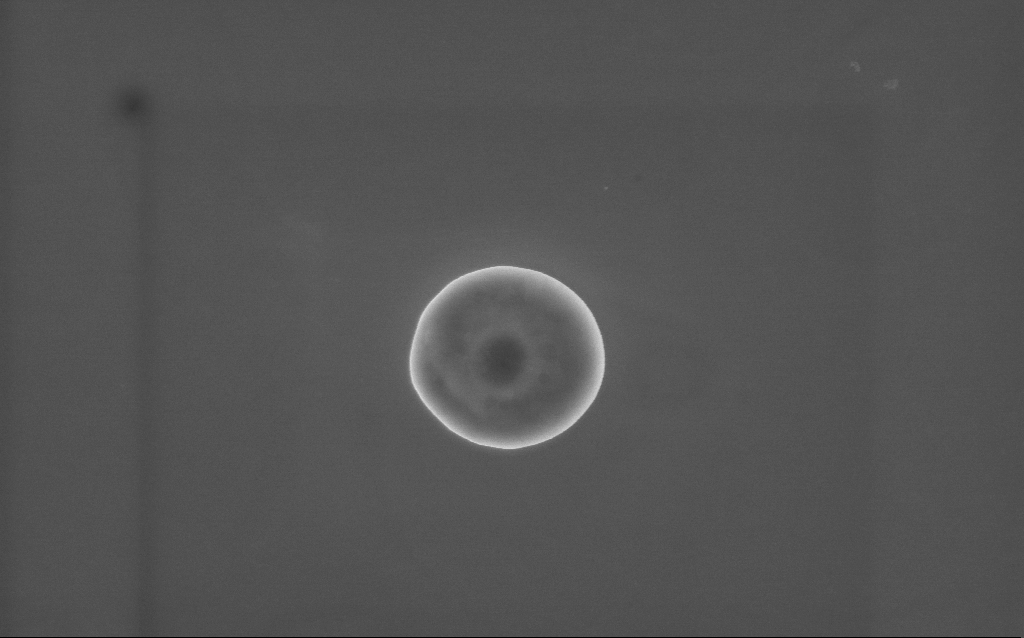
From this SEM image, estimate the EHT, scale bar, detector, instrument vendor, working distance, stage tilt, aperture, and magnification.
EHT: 10 kV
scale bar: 1000 nm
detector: InLens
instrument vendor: Zeiss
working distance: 2 mm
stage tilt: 0°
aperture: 30 µm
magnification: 57 K X